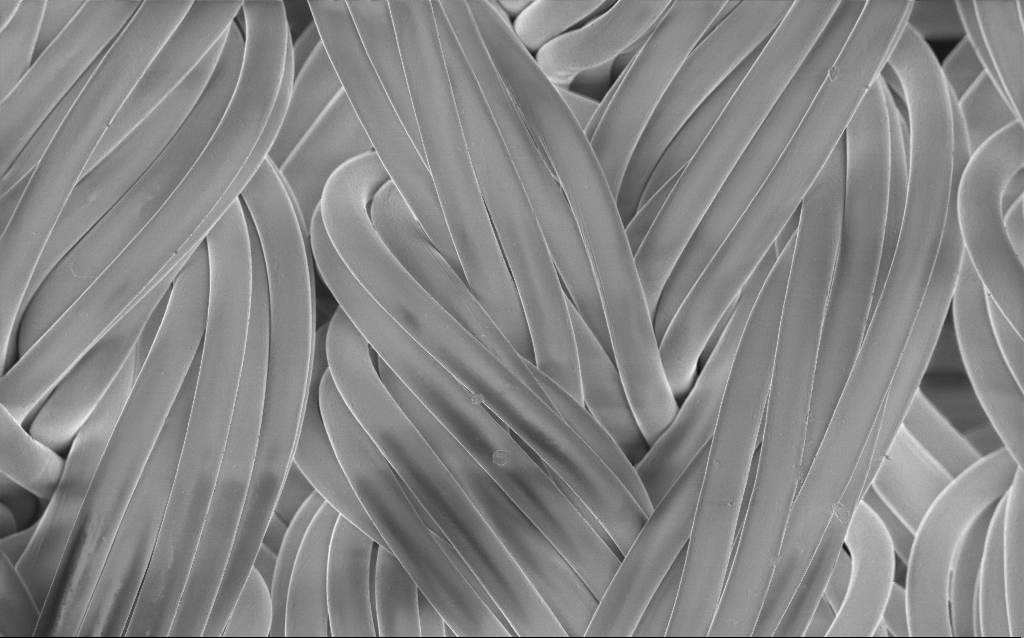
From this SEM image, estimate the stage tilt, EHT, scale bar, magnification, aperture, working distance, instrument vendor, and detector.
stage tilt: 0°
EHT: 1 kV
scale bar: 100000 nm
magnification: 0.656 K X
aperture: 30 µm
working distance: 4 mm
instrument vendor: Zeiss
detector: InLens